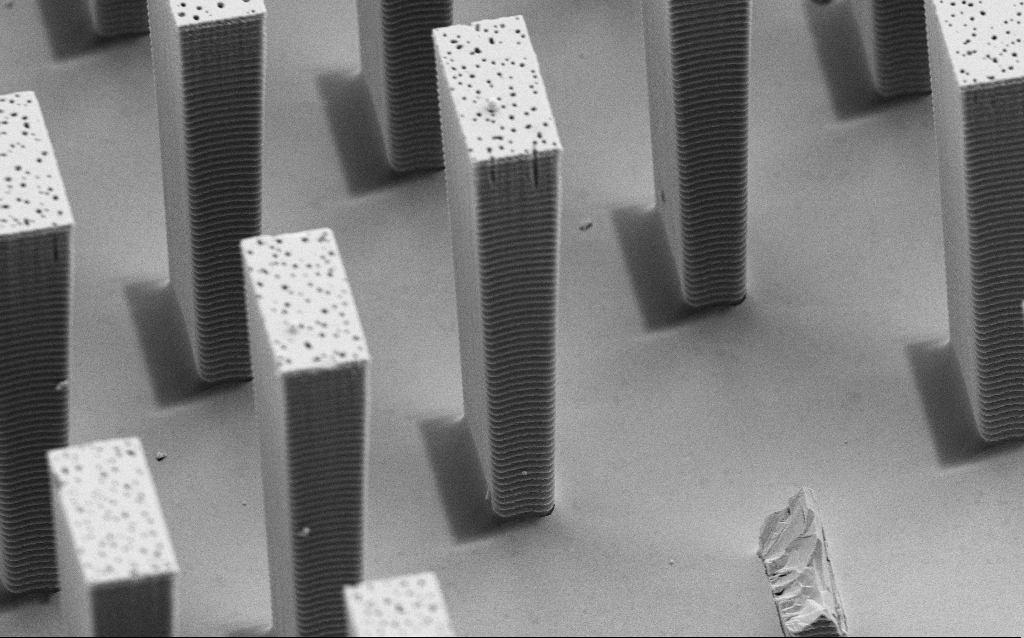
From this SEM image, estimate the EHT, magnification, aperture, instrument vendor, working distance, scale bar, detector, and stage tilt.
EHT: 5 kV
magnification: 9.09 K X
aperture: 30 µm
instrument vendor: Zeiss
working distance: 8 mm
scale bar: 2000 nm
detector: SE2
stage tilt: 45°